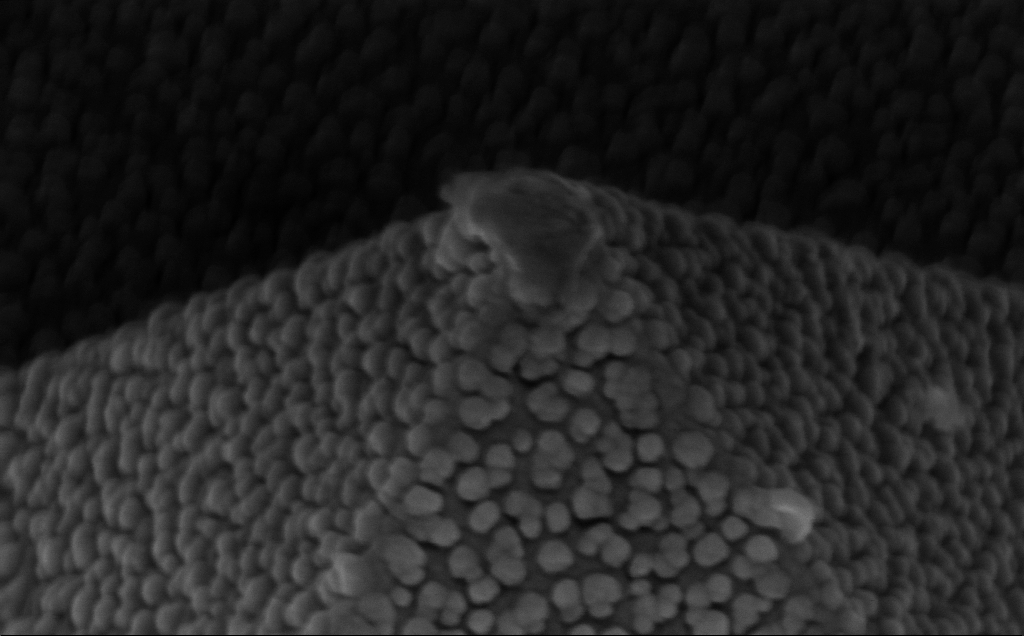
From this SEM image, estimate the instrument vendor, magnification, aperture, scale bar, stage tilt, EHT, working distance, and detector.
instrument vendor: Zeiss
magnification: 165.86 K X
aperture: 30 µm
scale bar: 200 nm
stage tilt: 45°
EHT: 10 kV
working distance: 8 mm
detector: InLens